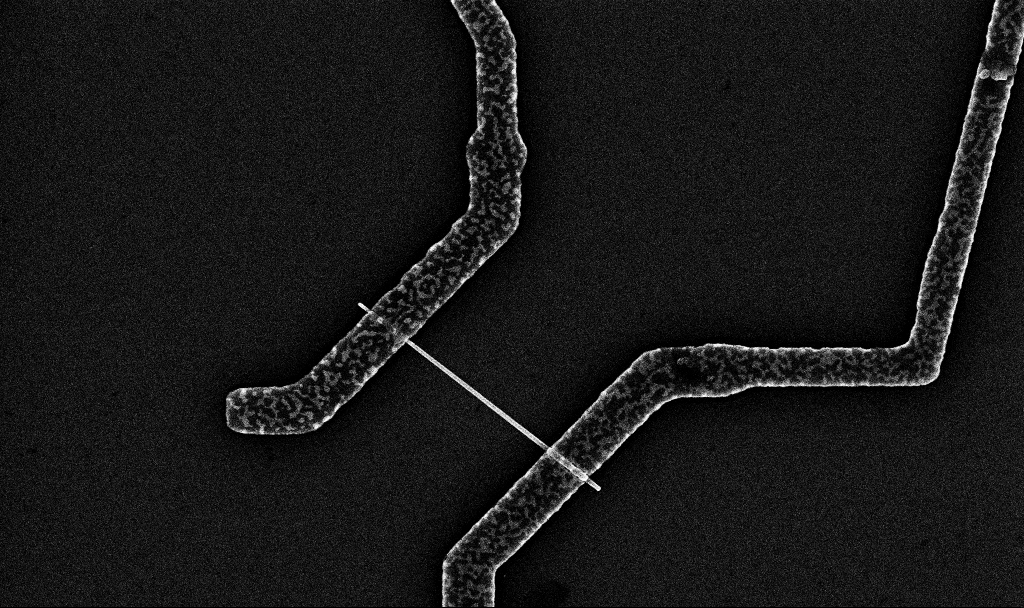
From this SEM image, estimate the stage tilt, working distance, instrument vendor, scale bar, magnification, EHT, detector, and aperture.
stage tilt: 0°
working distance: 6.7 mm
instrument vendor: Zeiss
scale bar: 1000 nm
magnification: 20 K X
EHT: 10 kV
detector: InLens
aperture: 30 µm